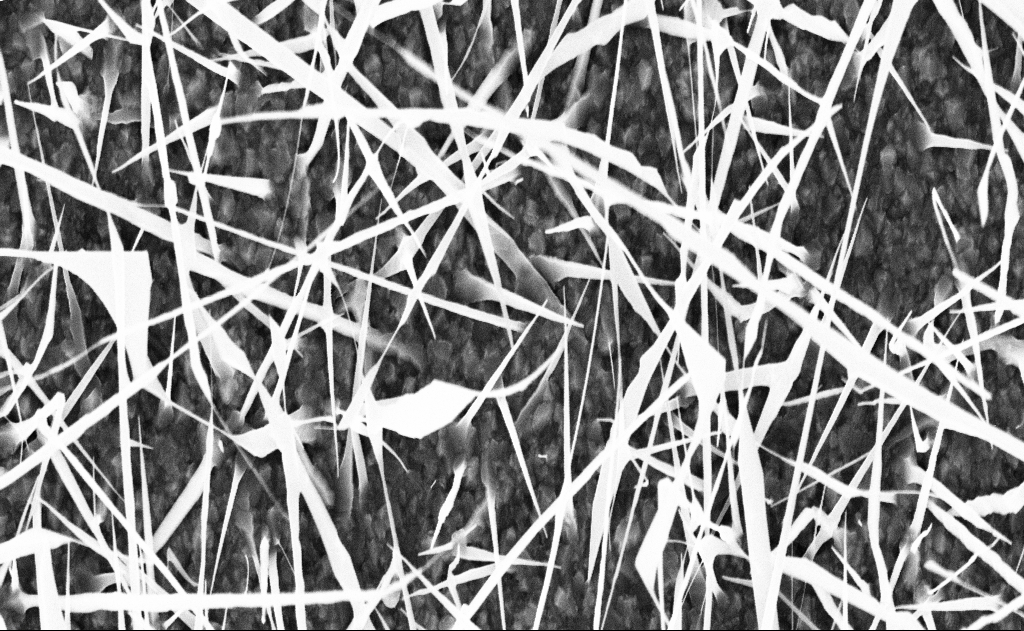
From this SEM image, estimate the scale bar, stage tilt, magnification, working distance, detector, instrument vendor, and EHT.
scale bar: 1000 nm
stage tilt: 0°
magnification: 20 K X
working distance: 17 mm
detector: InLens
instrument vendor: Zeiss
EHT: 10 kV